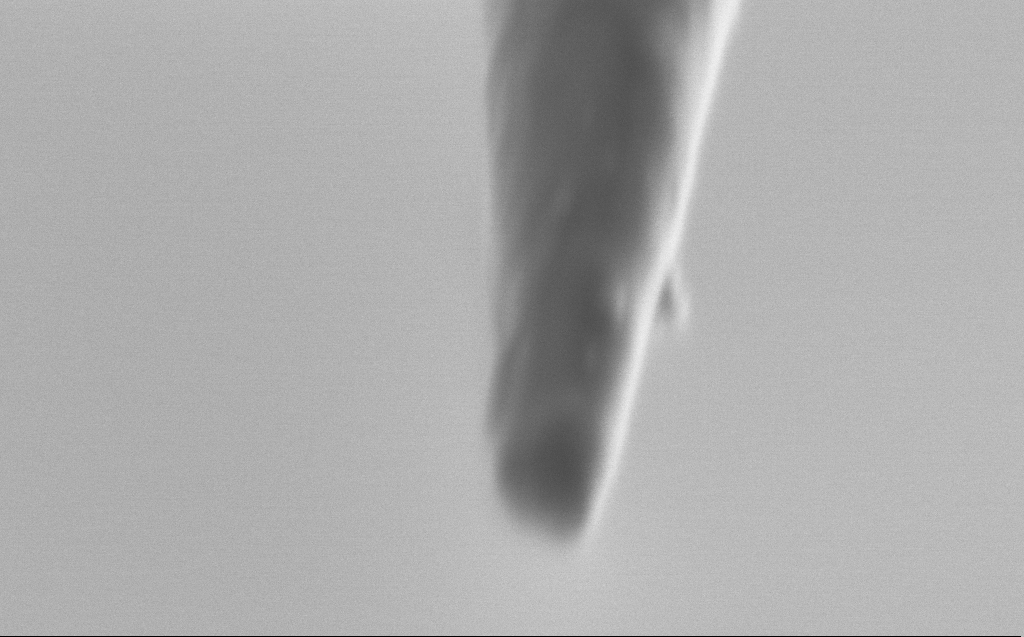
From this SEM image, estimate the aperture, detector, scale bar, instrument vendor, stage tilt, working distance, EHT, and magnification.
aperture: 30 µm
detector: SE2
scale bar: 200 nm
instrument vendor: Zeiss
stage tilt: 45°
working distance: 5 mm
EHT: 1 kV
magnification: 250 K X